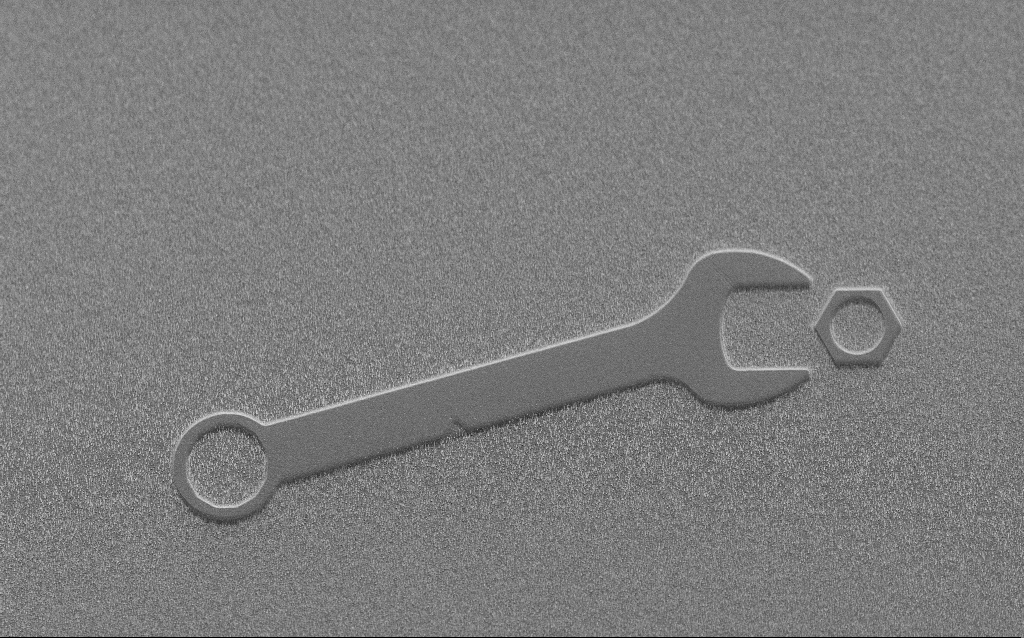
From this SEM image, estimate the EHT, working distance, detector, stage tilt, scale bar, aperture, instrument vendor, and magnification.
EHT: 5 kV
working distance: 8 mm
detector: SE2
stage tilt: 45°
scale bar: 100000 nm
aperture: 30 µm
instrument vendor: Zeiss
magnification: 0.59 K X